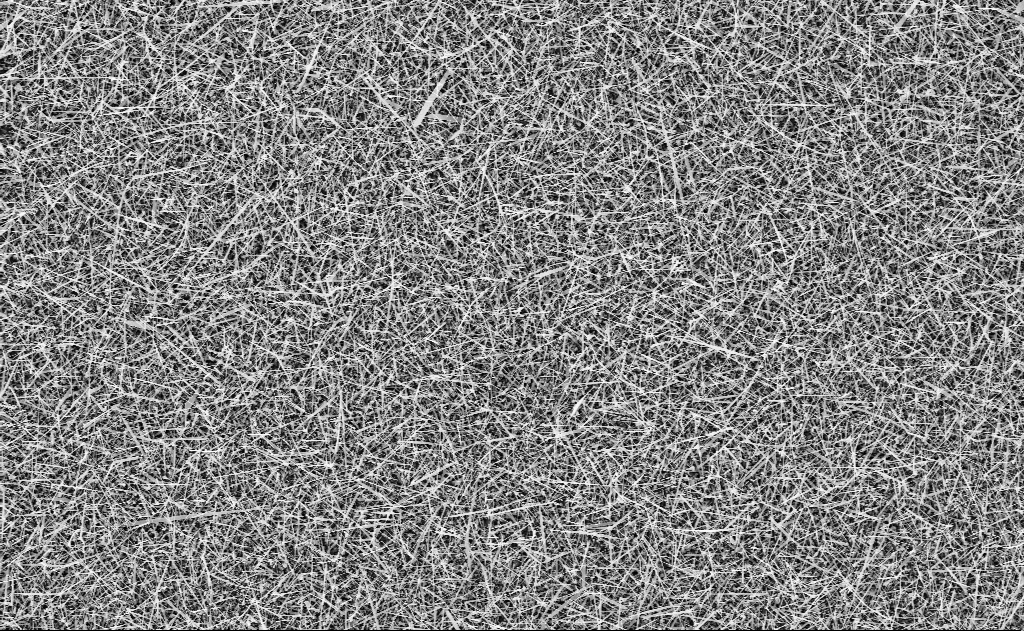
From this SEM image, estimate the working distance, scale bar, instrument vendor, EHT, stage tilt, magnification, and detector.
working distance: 11 mm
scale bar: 10000 nm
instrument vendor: Zeiss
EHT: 10 kV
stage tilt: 0°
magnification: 5 K X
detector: InLens